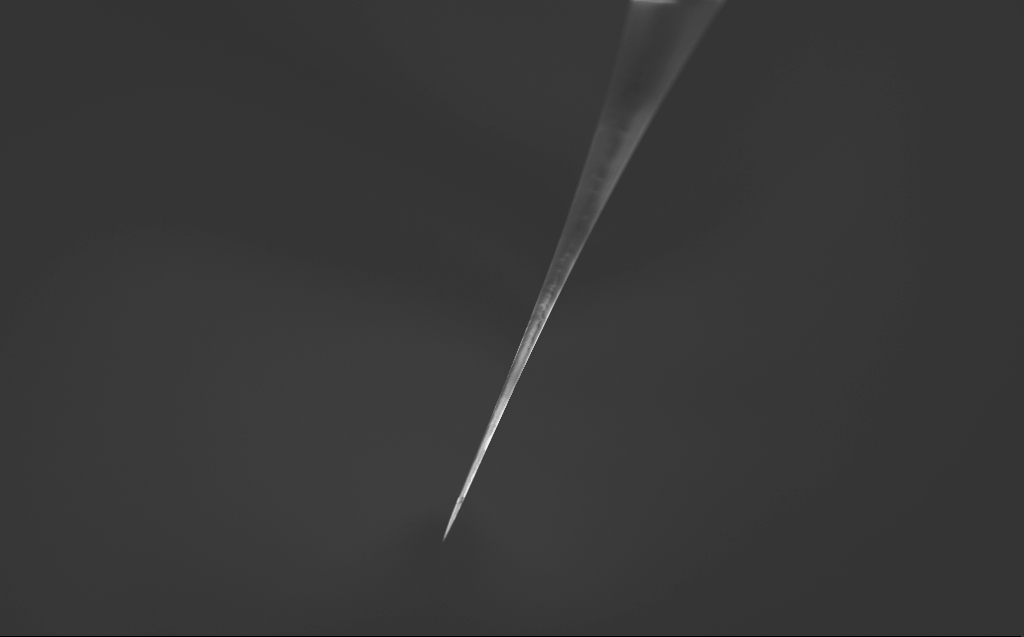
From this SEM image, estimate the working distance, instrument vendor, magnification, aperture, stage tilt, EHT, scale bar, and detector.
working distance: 3 mm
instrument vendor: Zeiss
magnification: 0.1 K X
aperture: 30 µm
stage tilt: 45°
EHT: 2 kV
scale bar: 200000 nm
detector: InLens